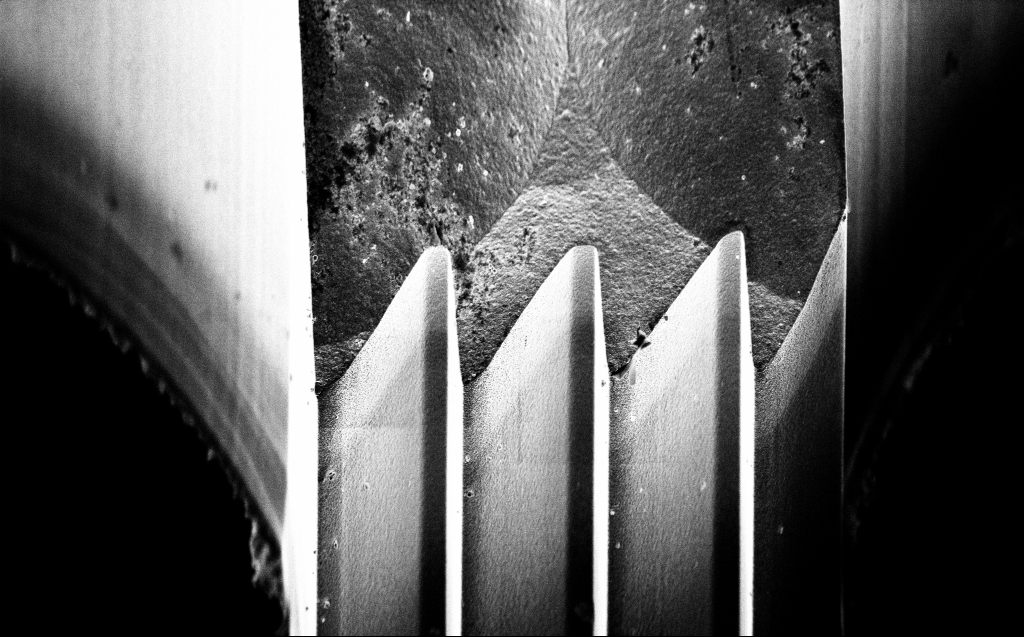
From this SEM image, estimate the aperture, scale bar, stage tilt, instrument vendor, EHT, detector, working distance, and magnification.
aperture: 30 µm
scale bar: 10000 nm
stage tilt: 45°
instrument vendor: Zeiss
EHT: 5 kV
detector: InLens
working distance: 5 mm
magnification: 4.78 K X